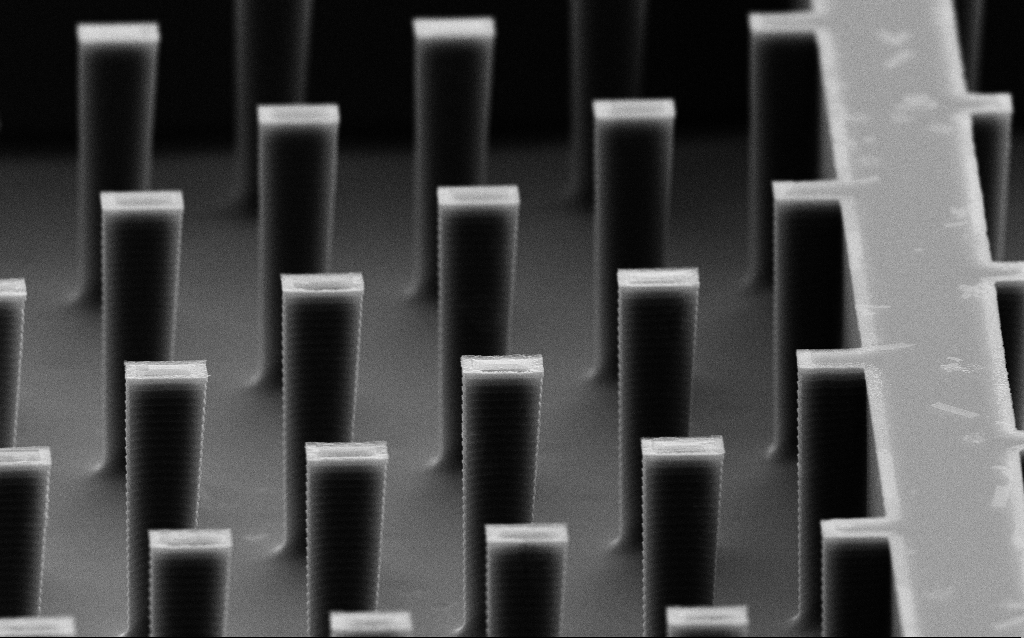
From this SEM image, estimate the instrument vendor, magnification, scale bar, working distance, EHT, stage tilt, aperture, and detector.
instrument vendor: Zeiss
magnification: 10.25 K X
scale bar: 2000 nm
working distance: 7.4 mm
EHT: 10 kV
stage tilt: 70°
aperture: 30 µm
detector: SE2